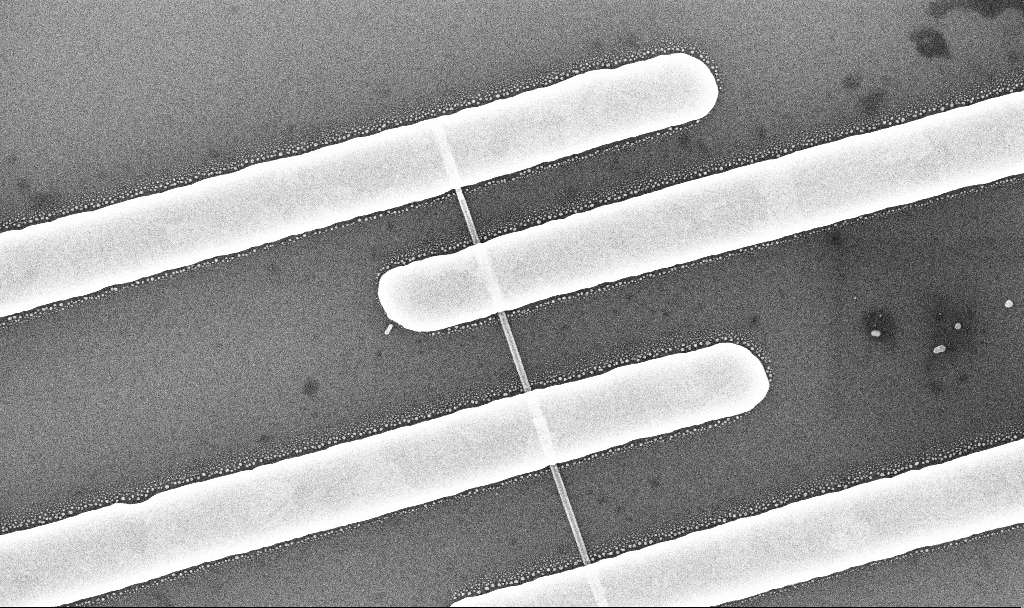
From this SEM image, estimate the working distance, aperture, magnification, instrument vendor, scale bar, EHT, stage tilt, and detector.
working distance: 7 mm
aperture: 30 µm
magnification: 65.26 K X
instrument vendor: Zeiss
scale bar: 1000 nm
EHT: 10 kV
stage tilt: -0°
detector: InLens